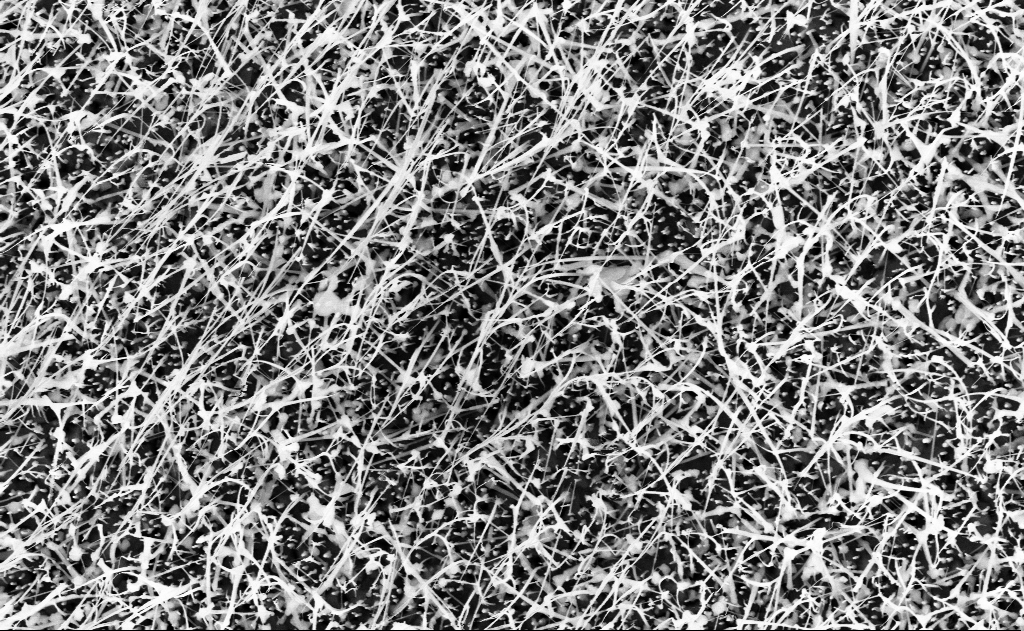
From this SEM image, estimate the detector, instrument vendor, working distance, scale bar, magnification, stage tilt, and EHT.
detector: InLens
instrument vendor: Zeiss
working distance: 14 mm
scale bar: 1000 nm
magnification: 20 K X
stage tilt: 0°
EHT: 10 kV